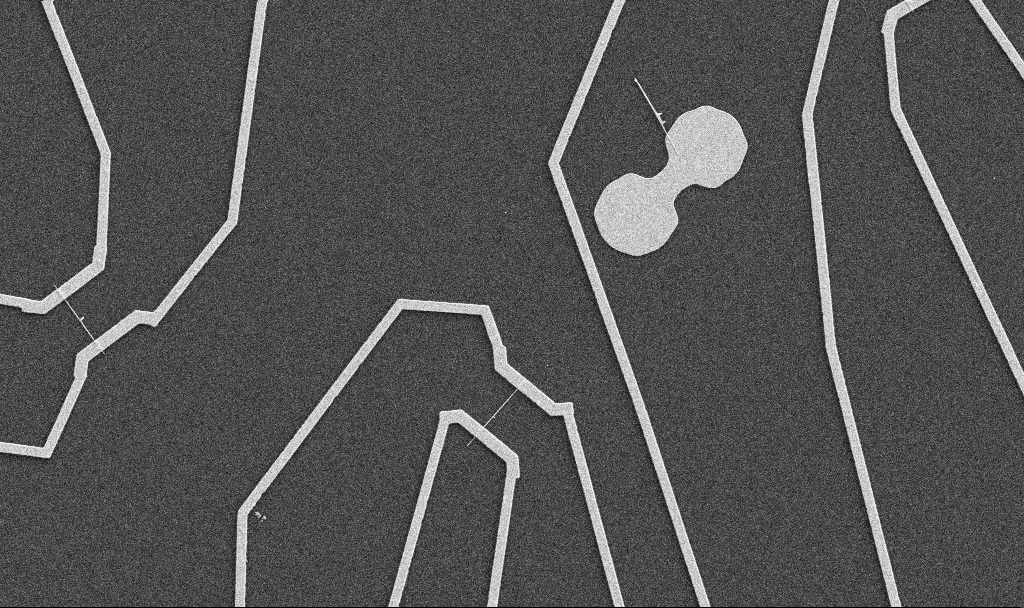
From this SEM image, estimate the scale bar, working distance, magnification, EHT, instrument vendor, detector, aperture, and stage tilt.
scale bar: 10000 nm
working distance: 10.7 mm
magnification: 5 K X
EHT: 5 kV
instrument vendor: Zeiss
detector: SE2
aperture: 30 µm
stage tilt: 0°